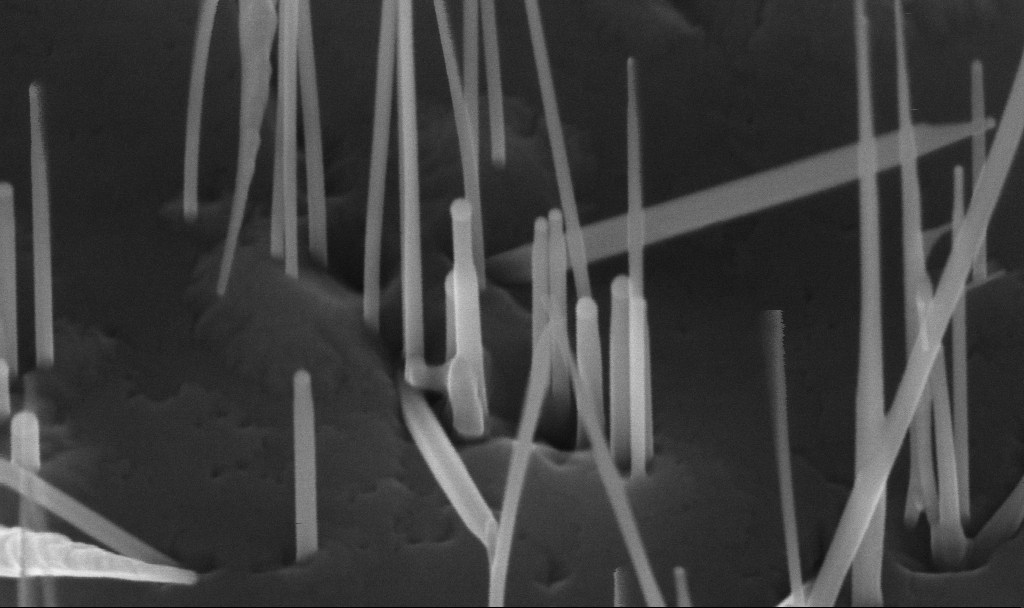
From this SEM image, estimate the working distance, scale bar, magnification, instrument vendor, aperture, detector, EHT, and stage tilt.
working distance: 5.6 mm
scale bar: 100 nm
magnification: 200 K X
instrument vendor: Zeiss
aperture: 30 µm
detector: InLens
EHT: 10 kV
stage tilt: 45°